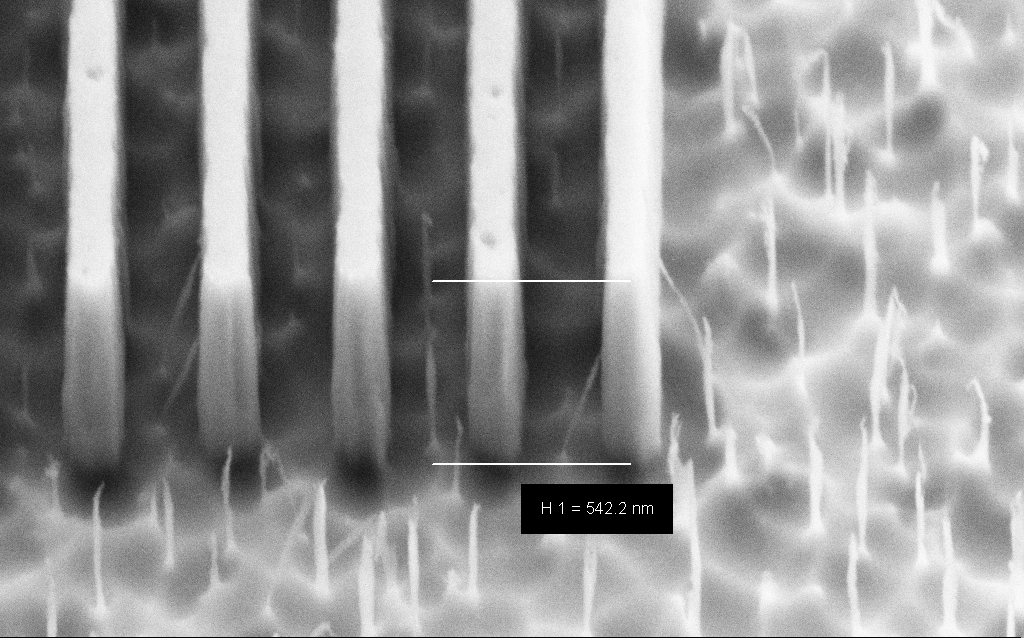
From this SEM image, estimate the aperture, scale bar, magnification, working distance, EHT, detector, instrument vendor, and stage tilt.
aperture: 30 µm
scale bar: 200 nm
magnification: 123.94 K X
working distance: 5 mm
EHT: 3 kV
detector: SE2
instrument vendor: Zeiss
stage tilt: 45°